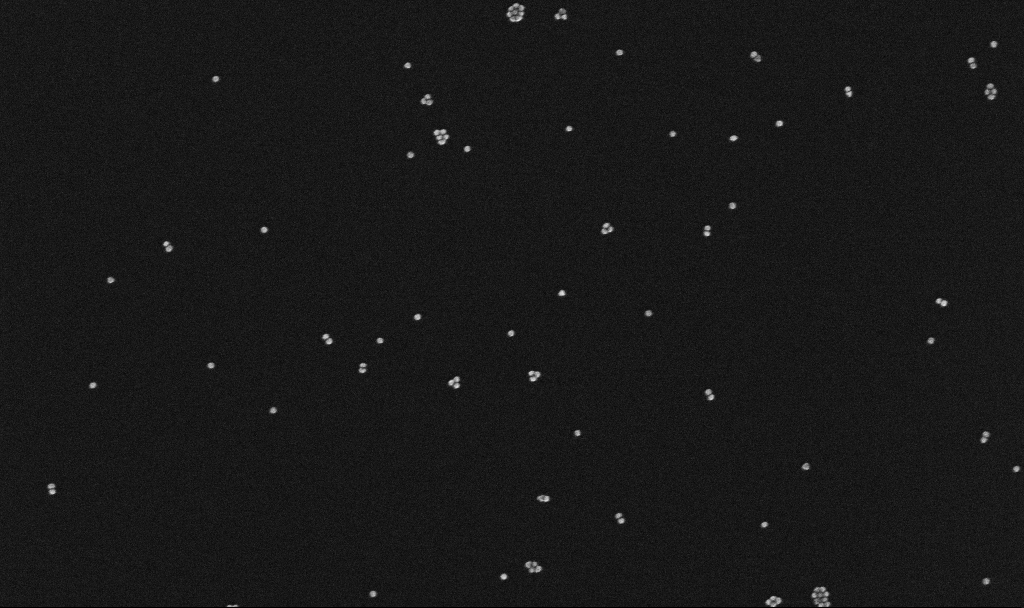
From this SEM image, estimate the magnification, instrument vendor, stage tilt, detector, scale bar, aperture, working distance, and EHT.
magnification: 100 K X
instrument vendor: Zeiss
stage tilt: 0°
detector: InLens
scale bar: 200 nm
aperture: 30 µm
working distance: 3.2 mm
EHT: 10 kV